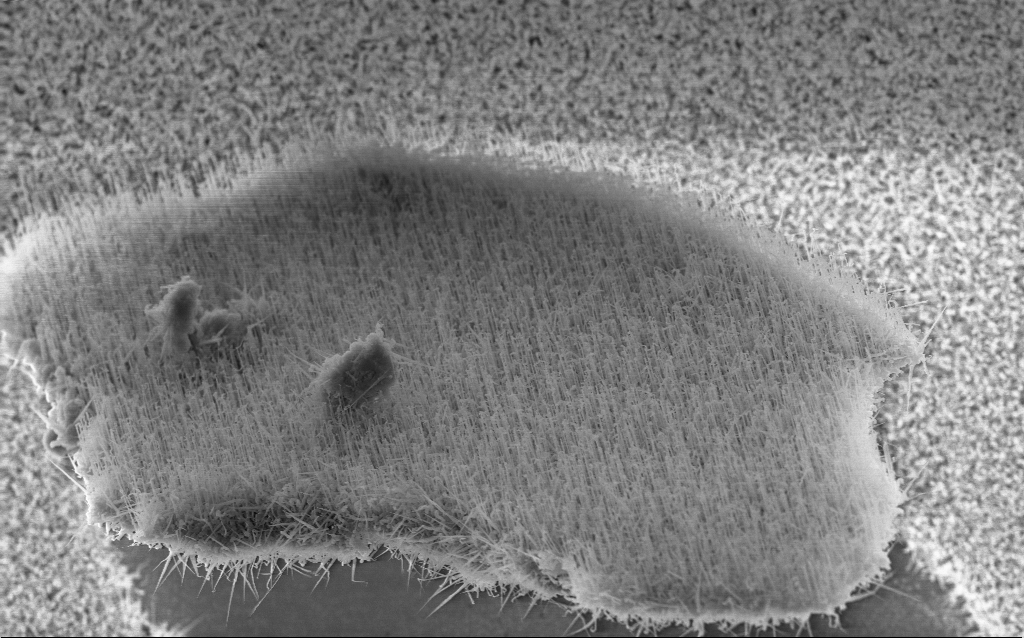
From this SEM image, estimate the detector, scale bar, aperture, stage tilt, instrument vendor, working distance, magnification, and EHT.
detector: InLens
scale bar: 1000 nm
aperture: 30 µm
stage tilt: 0°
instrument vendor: Zeiss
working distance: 7.6 mm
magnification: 12.66 K X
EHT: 10 kV